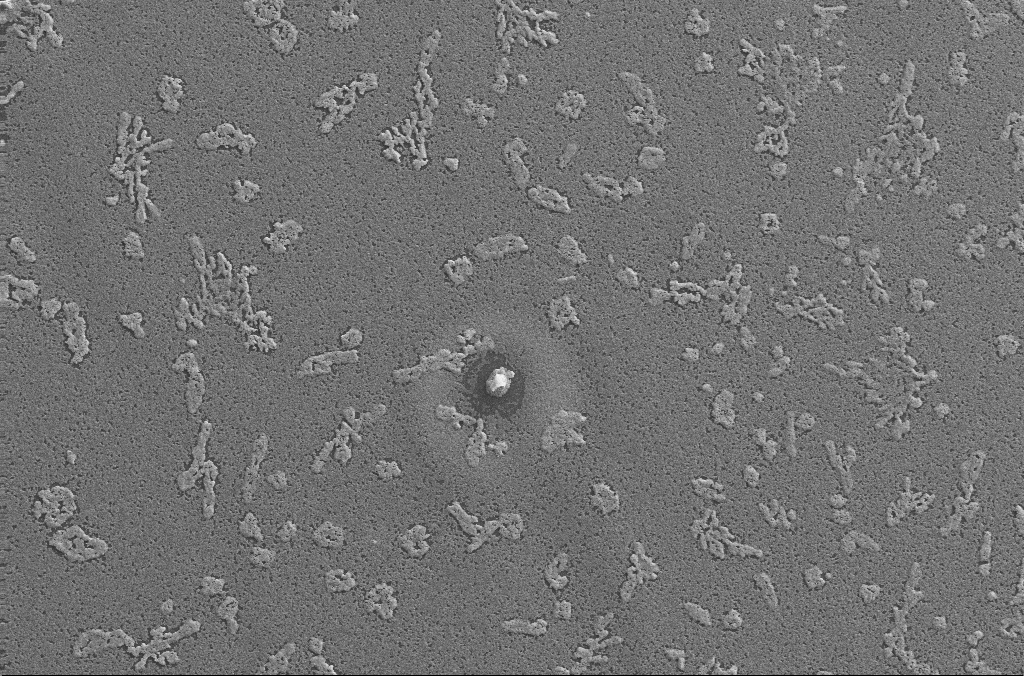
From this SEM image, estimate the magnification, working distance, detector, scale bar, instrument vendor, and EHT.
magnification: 0.5 K X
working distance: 2.9 mm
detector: SE2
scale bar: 100000 nm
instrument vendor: Zeiss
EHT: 20 kV